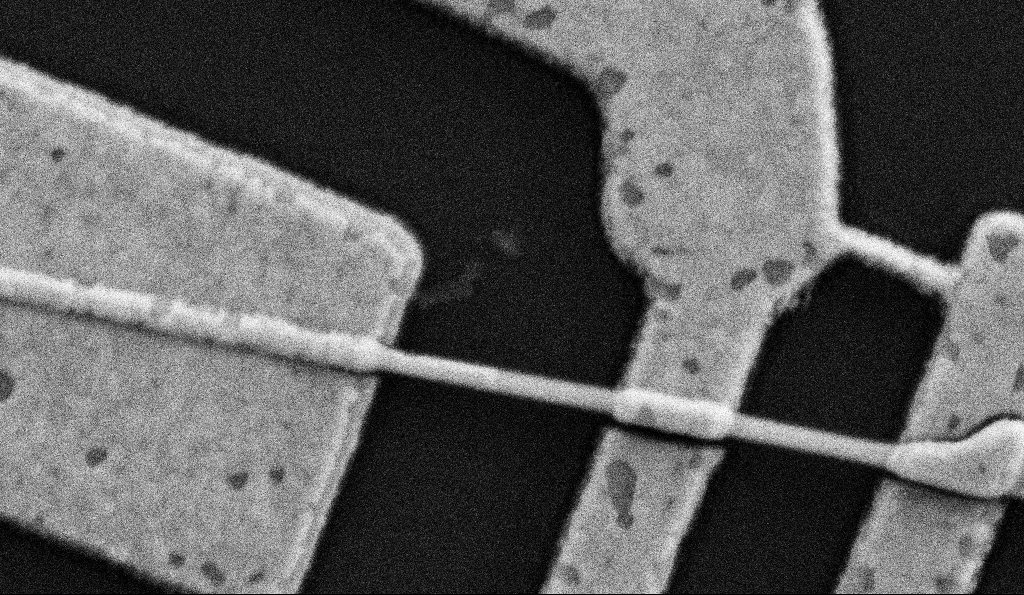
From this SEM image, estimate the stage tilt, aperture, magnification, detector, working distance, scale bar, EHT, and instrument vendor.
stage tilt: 0°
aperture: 30 µm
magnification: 100 K X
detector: SE2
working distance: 8.5 mm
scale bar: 200 nm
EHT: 5 kV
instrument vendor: Zeiss